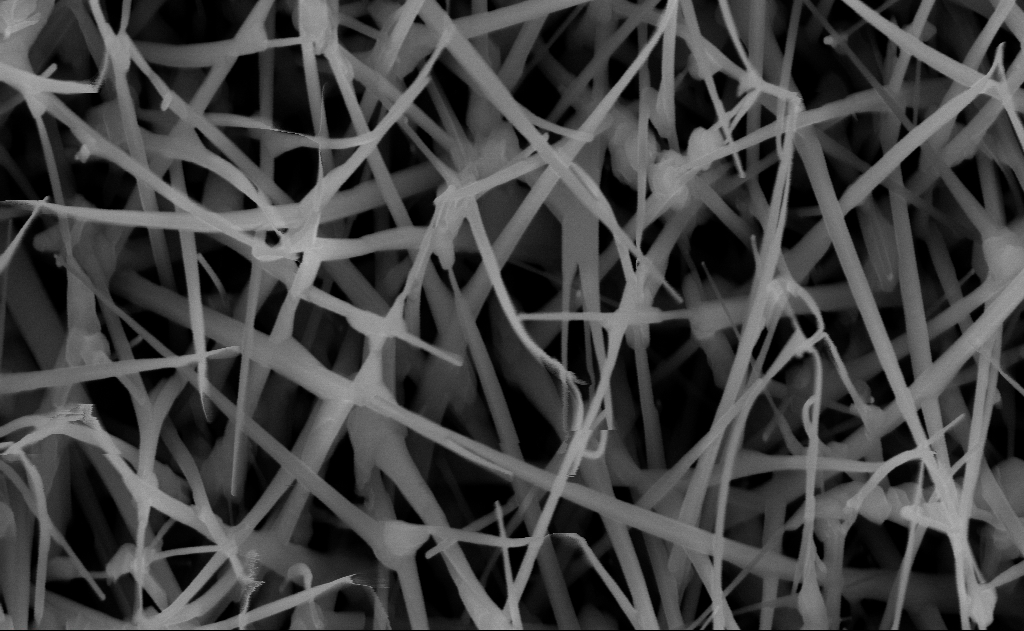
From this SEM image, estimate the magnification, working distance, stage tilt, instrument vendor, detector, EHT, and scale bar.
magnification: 80 K X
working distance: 10 mm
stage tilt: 0°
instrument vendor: Zeiss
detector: InLens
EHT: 10 kV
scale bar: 200 nm